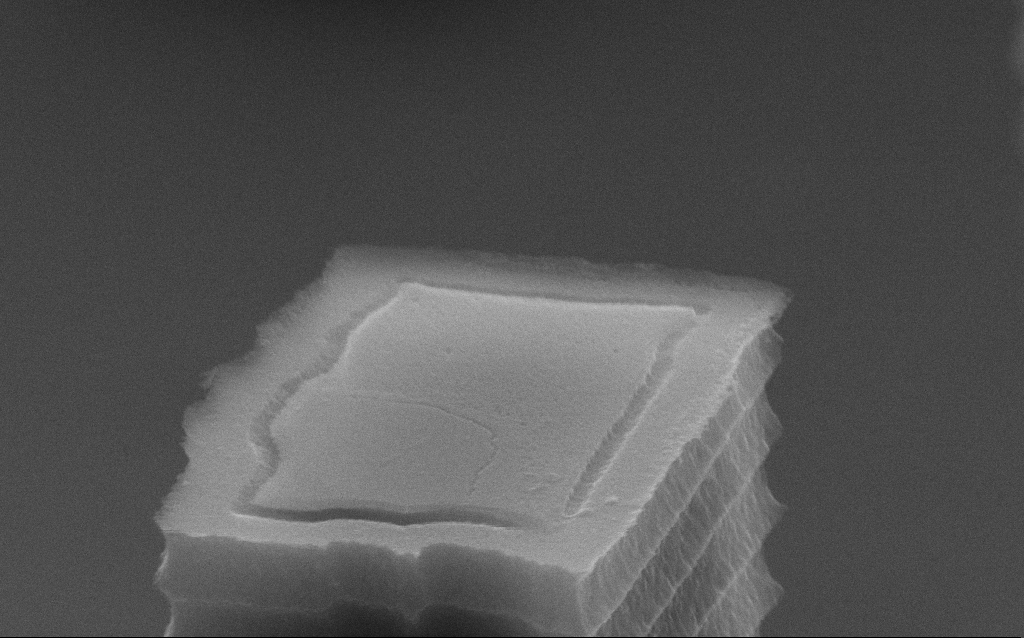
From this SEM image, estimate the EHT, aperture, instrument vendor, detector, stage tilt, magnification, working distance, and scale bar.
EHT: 10 kV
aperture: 30 µm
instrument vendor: Zeiss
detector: SE2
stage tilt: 70°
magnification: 93.52 K X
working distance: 7.2 mm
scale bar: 200 nm